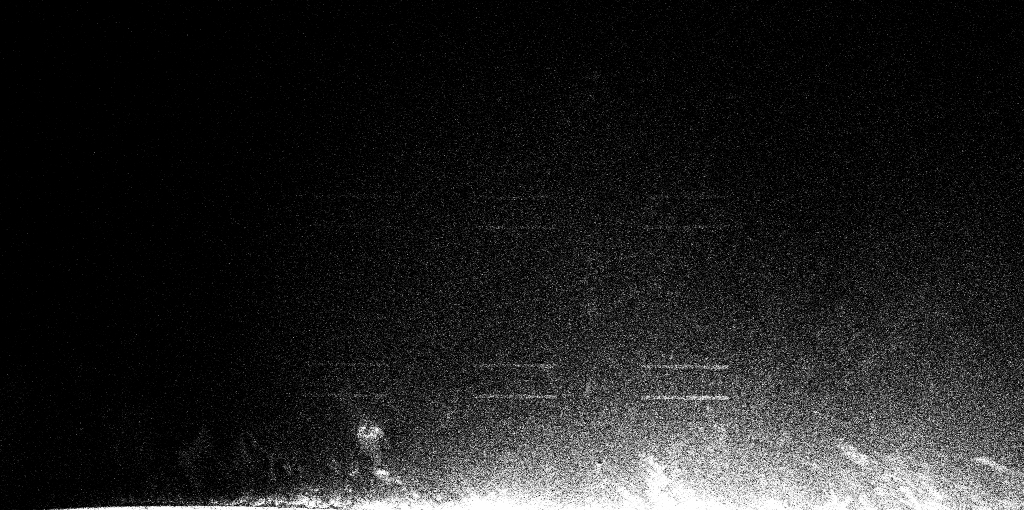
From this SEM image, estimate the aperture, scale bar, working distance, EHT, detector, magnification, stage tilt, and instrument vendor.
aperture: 30 µm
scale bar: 1e+06 nm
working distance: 9.7 mm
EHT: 5 kV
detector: InLens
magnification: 0.064 K X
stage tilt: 45°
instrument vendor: Zeiss